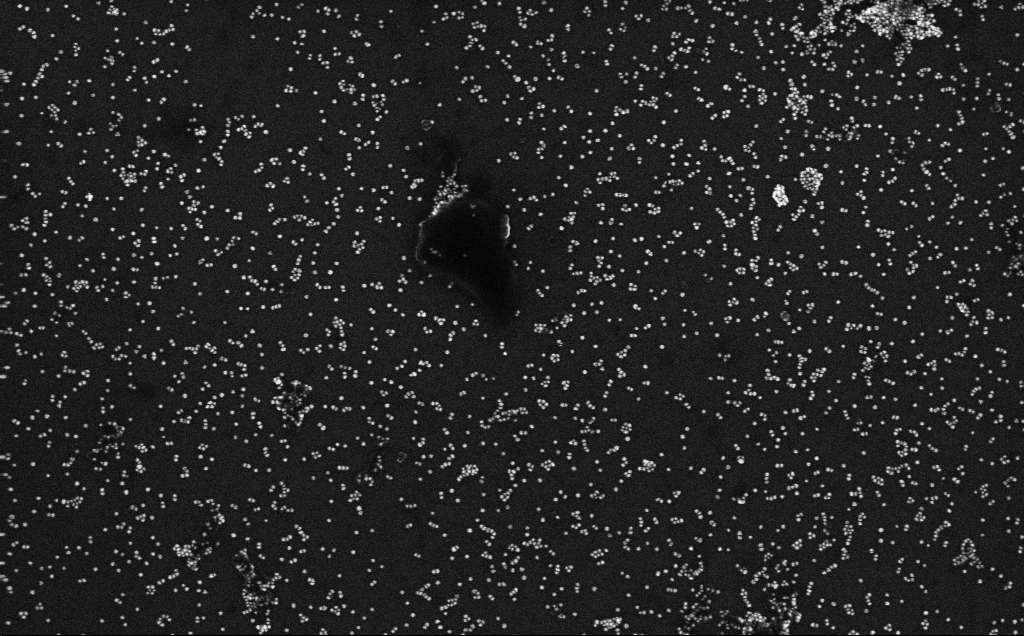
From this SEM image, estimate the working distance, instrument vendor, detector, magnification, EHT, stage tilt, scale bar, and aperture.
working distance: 3.3 mm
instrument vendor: Zeiss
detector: InLens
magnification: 100 K X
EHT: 10 kV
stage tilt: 0°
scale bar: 200 nm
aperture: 30 µm